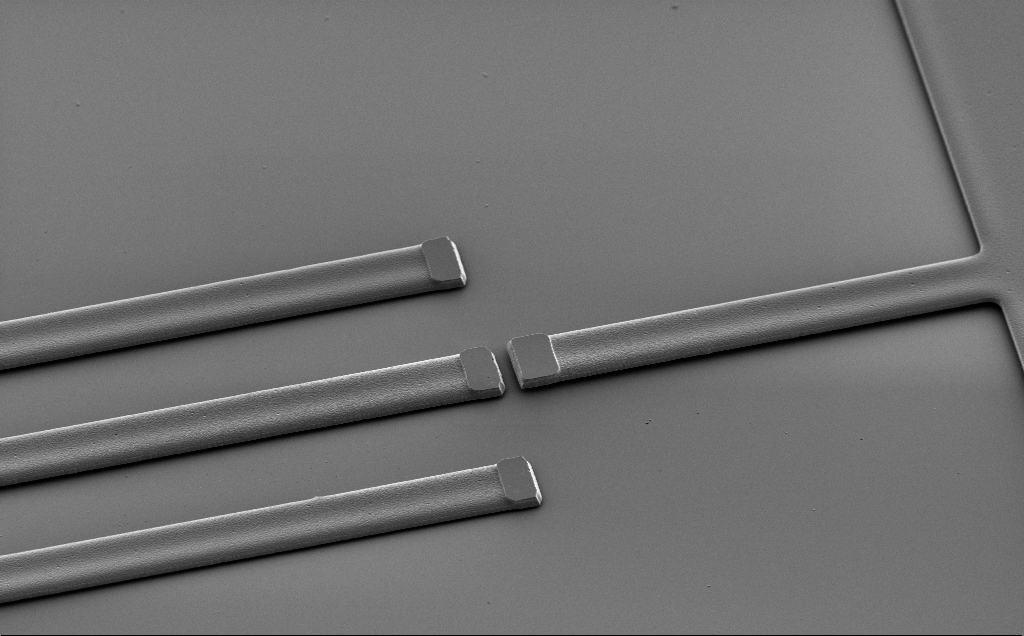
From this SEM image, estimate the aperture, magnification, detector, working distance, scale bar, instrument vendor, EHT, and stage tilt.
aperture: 30 µm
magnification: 1.43 K X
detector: SE2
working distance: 10 mm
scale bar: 10000 nm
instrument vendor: Zeiss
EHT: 2 kV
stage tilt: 43°